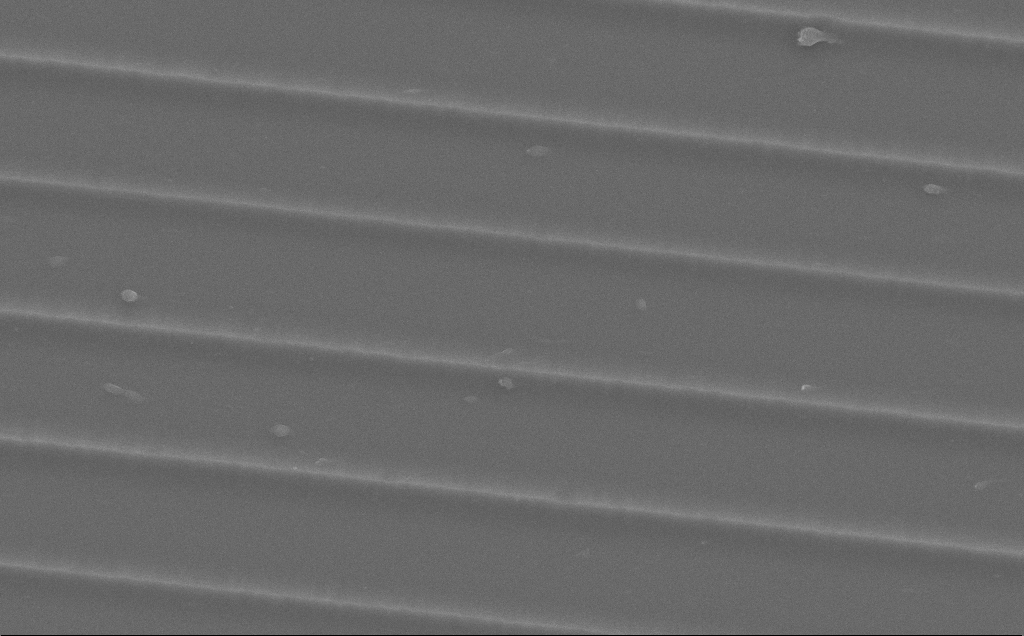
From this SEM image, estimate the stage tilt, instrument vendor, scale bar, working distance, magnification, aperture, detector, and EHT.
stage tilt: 0°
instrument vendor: Zeiss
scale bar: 10000 nm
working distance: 11 mm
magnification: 6.94 K X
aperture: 30 µm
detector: SE2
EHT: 10 kV